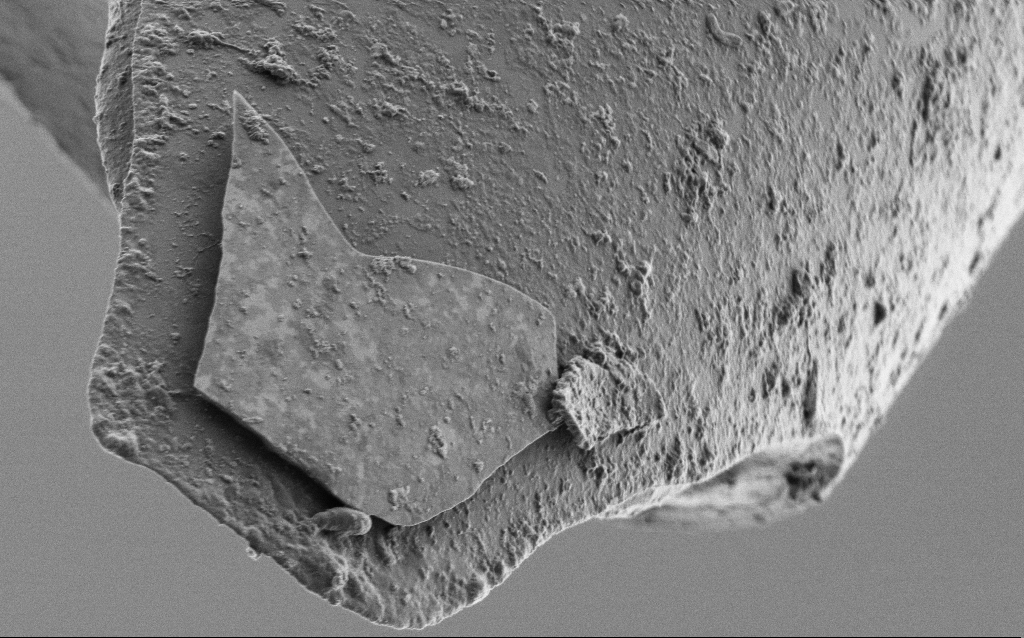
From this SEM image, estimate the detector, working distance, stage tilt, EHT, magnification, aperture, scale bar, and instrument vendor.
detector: SE2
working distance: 7.7 mm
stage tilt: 45°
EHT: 1 kV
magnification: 10 K X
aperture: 30 µm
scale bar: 2000 nm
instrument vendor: Zeiss